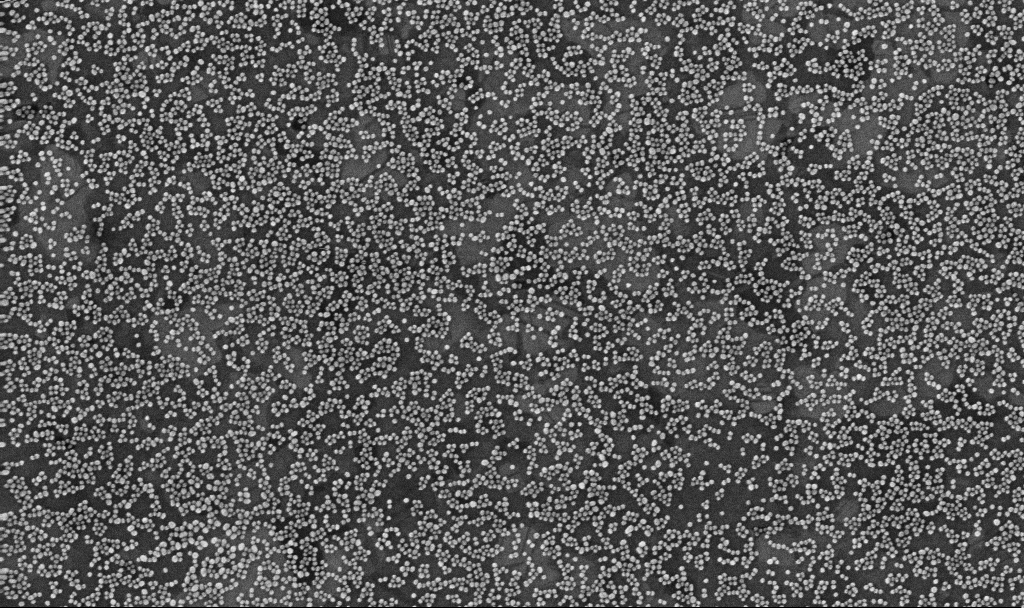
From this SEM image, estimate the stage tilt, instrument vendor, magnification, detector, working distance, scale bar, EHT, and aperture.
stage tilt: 0°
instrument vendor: Zeiss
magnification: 100 K X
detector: InLens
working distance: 3.3 mm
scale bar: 200 nm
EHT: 10 kV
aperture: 30 µm